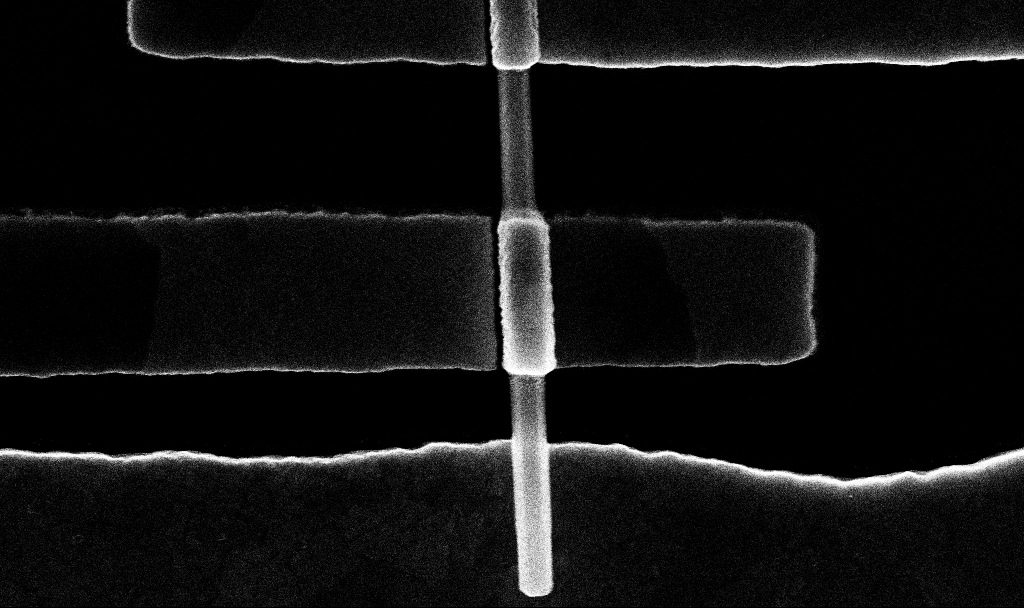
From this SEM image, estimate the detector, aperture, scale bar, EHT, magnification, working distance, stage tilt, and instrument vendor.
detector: InLens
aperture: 30 µm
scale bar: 200 nm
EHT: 10 kV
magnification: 98.91 K X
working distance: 6.8 mm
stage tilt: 0°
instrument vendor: Zeiss